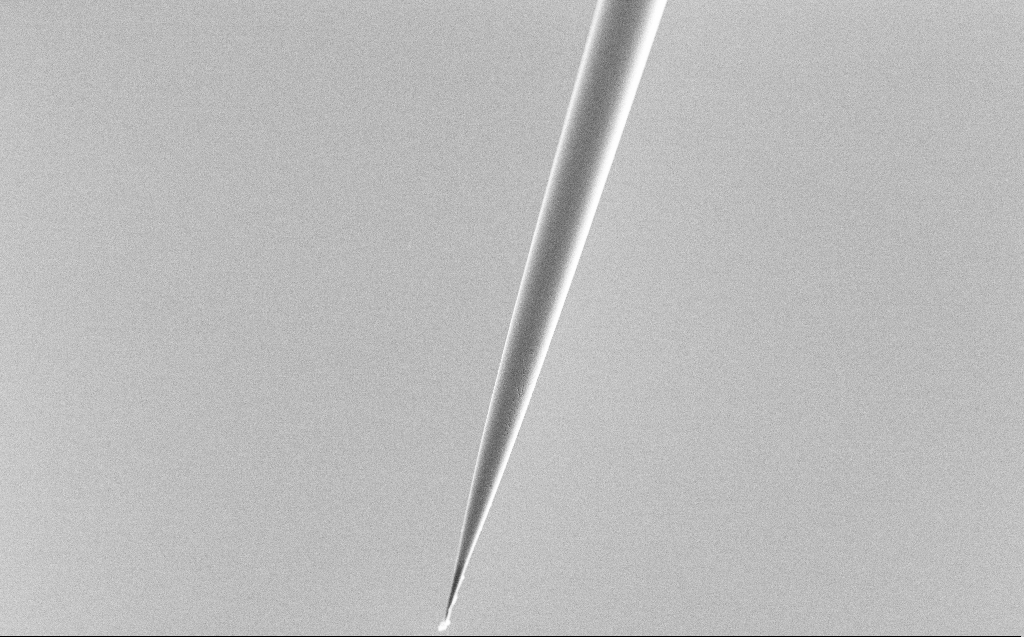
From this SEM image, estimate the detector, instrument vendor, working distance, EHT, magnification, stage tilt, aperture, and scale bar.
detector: SE2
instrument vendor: Zeiss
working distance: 6 mm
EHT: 5 kV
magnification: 1 K X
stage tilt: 45°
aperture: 30 µm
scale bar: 20000 nm